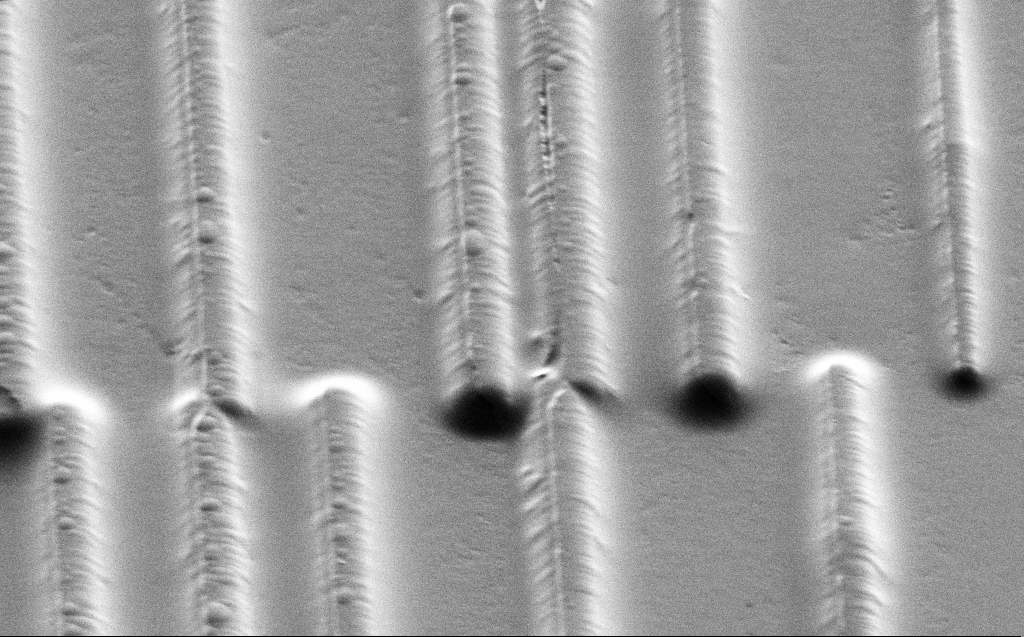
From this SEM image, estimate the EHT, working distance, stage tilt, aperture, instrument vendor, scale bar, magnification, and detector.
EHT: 3 kV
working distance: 11 mm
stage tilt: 45°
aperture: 30 µm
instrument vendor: Zeiss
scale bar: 2000 nm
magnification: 7.58 K X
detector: SE2